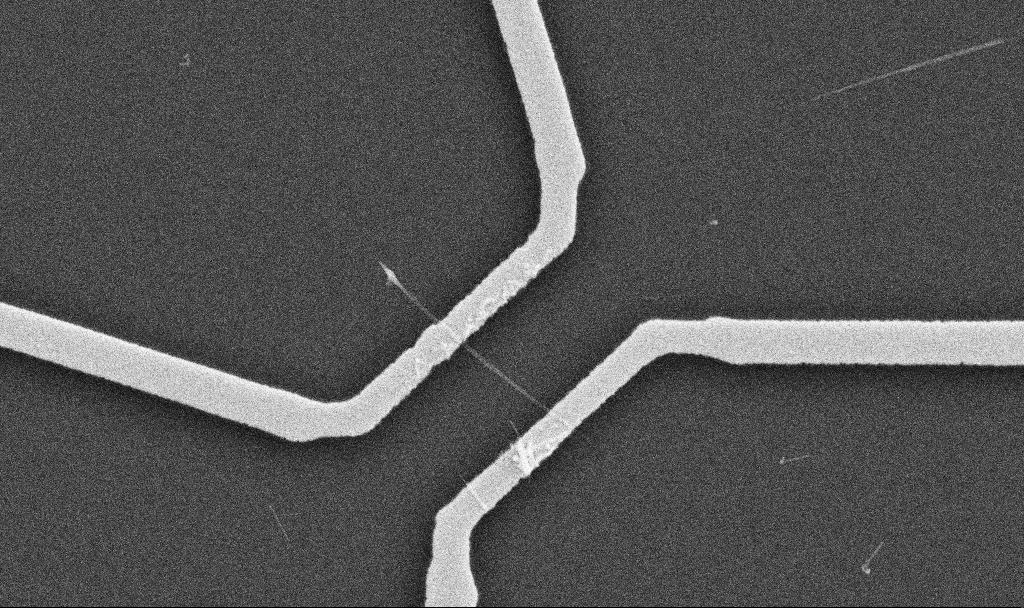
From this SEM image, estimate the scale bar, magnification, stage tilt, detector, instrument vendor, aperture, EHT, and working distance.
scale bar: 1000 nm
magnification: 20 K X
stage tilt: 0°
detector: SE2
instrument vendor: Zeiss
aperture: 30 µm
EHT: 10 kV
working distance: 10.7 mm